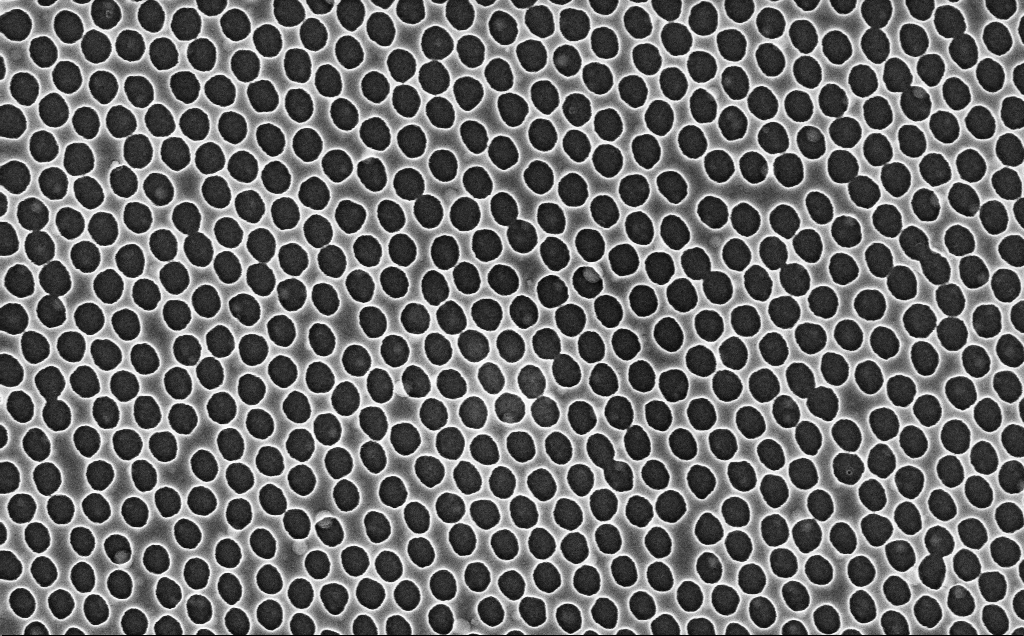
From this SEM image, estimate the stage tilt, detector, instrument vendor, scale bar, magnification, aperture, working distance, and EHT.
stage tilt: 0°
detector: InLens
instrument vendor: Zeiss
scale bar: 1000 nm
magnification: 39.86 K X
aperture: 30 µm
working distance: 2.4 mm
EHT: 3 kV